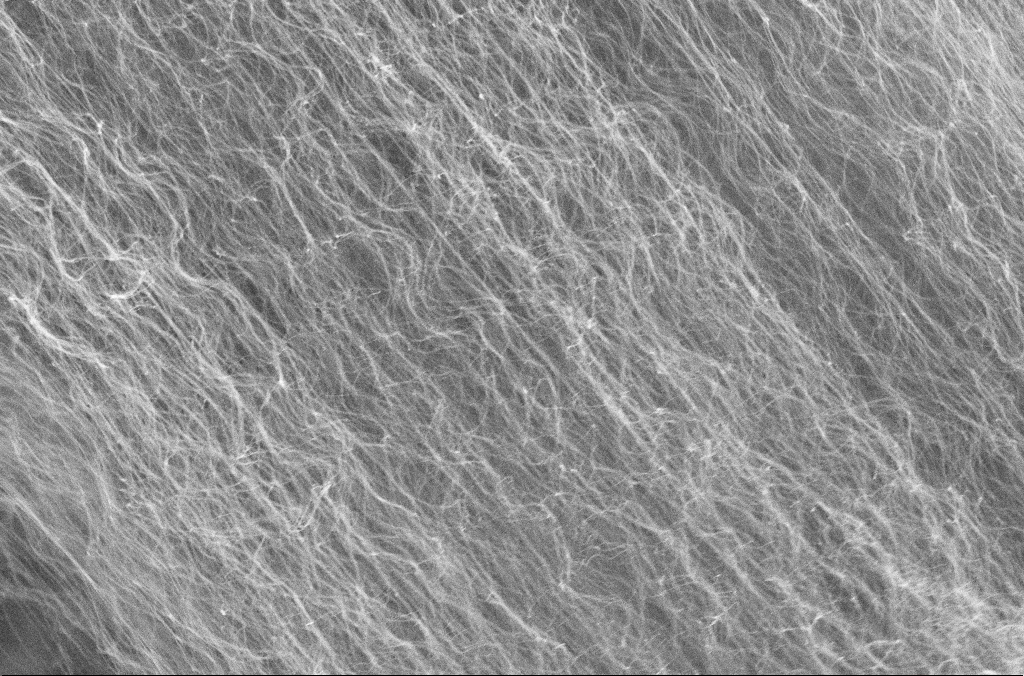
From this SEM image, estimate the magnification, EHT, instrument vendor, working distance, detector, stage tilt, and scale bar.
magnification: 50 K X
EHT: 10 kV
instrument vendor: Zeiss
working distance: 4 mm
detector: InLens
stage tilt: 0°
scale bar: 1000 nm